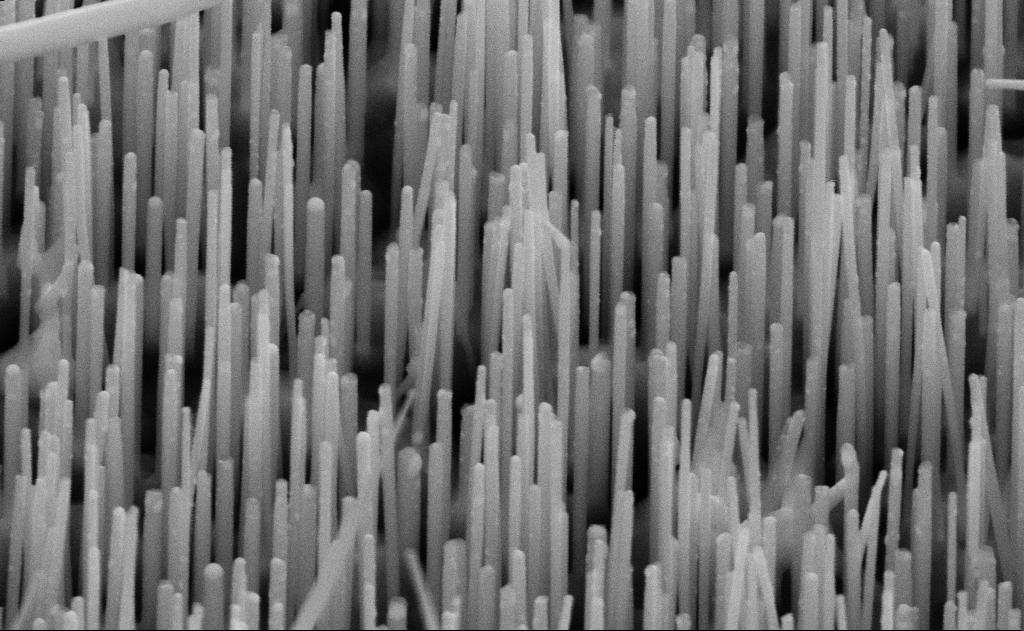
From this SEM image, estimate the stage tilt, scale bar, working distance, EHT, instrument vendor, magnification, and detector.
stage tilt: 45°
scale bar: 200 nm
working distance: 11 mm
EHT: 10 kV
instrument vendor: Zeiss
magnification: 100 K X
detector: SE2